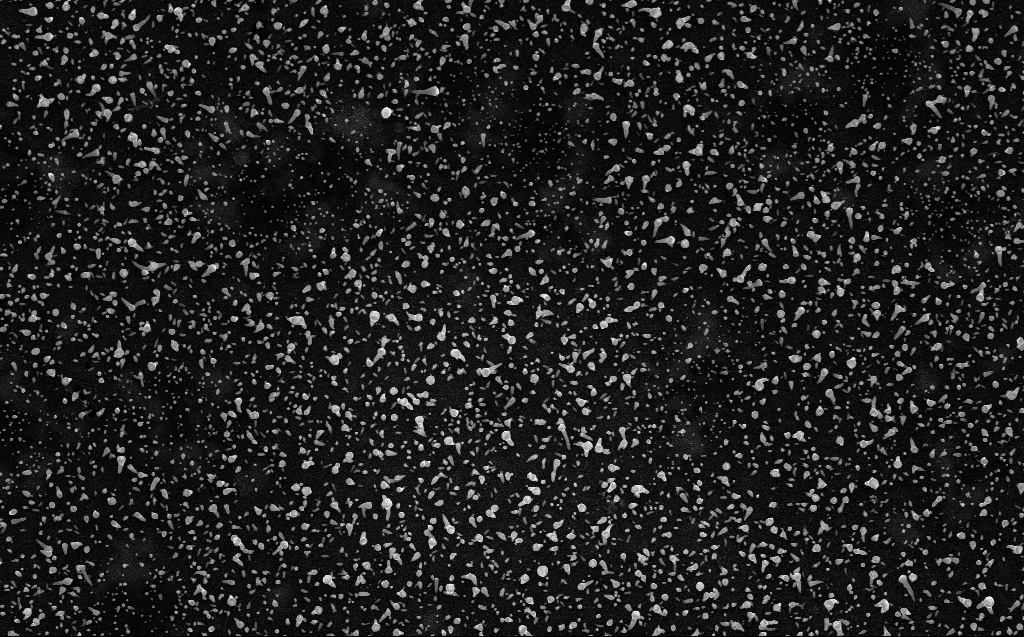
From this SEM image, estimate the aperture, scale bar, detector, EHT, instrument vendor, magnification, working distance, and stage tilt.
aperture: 30 µm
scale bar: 2000 nm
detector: InLens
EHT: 10 kV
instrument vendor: Zeiss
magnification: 13.68 K X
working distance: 3 mm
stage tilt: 0°